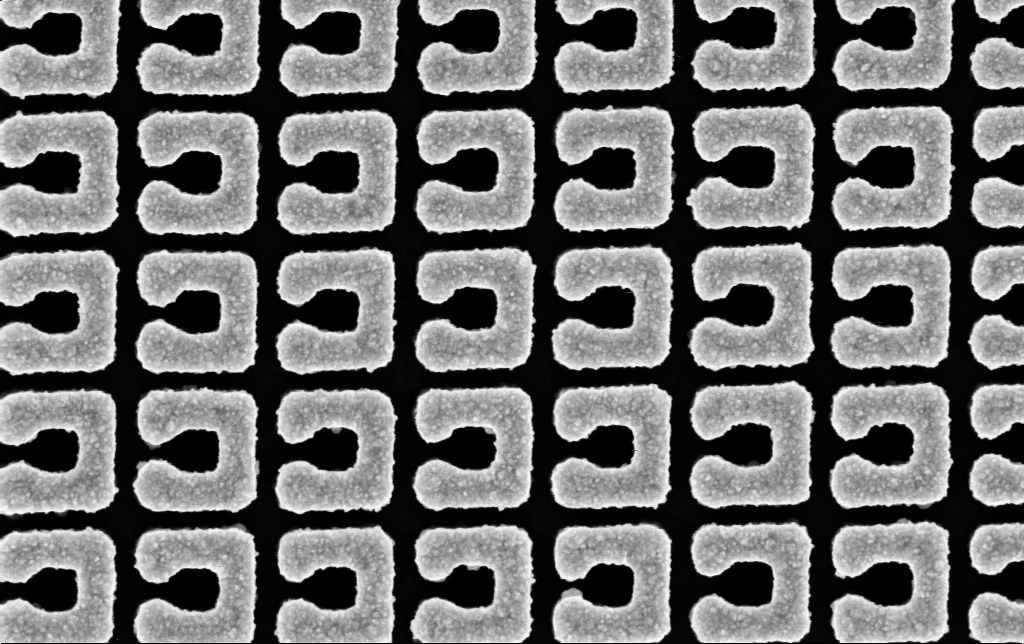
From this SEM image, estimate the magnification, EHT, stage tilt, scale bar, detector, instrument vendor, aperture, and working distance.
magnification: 111.37 K X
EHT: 5 kV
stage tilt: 0°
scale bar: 200 nm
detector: InLens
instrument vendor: Zeiss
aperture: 30 µm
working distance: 3.4 mm